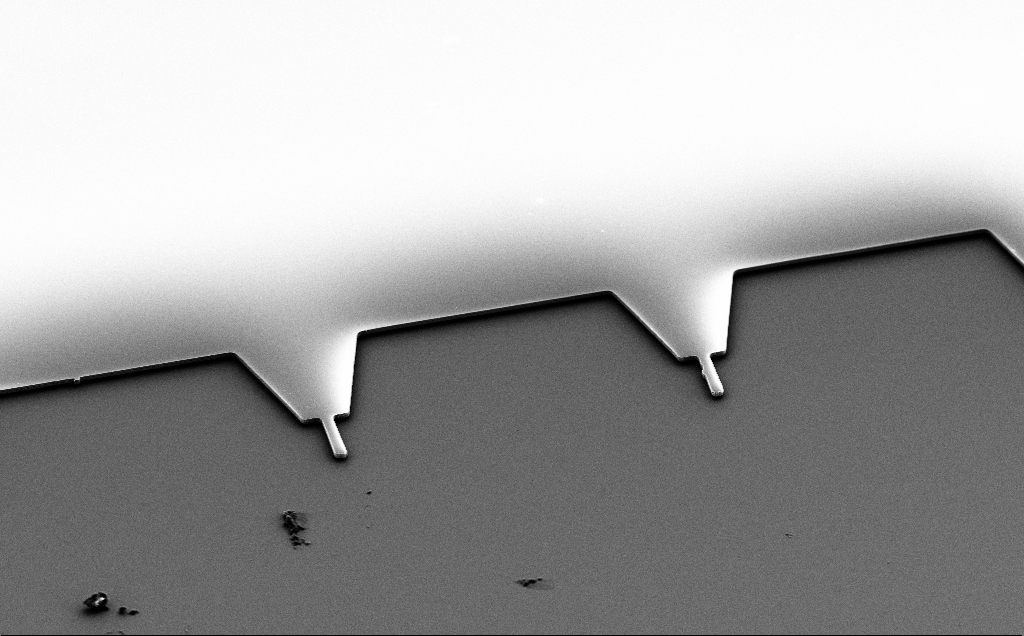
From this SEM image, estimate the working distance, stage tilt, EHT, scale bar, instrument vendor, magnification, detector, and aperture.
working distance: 10 mm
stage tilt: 50°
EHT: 5 kV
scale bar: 20000 nm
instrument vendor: Zeiss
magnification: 0.895 K X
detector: SE2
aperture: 30 µm